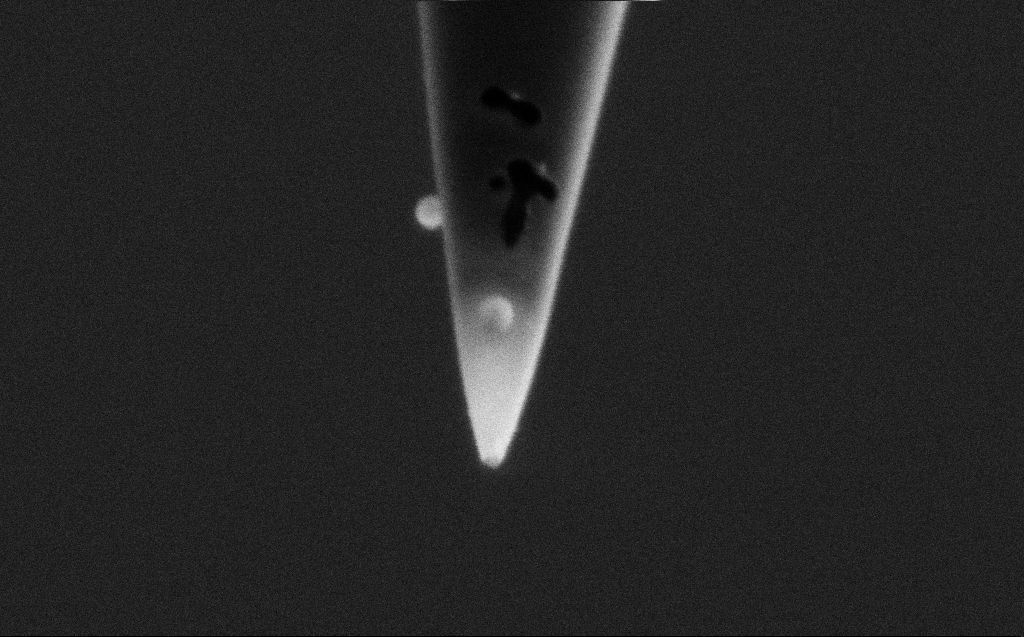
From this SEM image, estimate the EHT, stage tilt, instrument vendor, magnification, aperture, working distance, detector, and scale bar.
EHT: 2 kV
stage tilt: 45.1°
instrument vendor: Zeiss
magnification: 124.16 K X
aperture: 20 µm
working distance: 4 mm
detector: SE2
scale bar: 200 nm